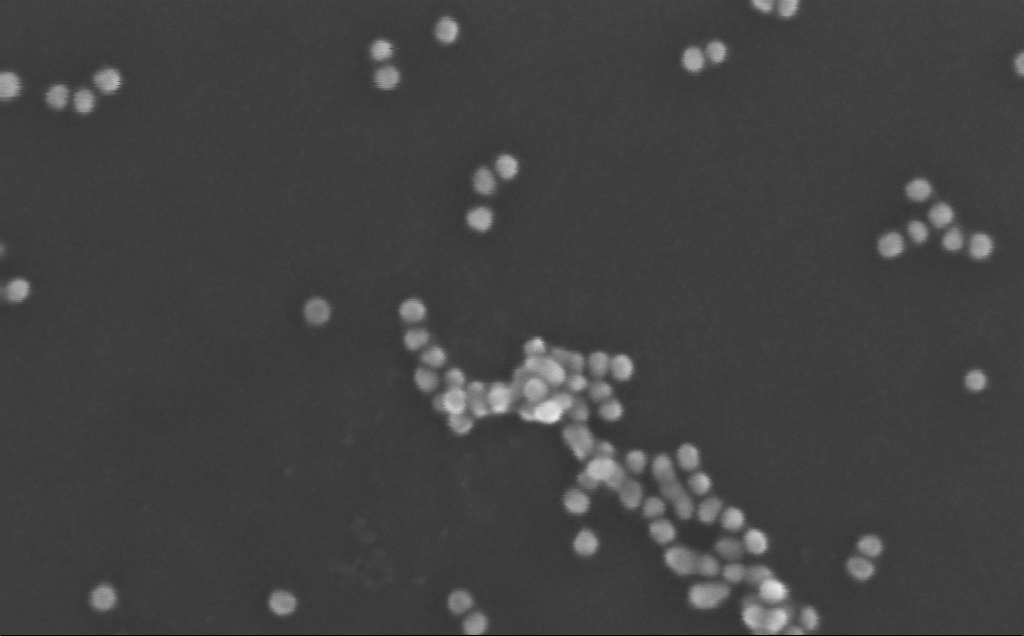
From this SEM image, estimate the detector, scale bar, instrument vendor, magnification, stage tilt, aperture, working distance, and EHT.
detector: InLens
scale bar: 100 nm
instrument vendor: Zeiss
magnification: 547.44 K X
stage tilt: -0°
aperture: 30 µm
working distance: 3 mm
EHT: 10 kV